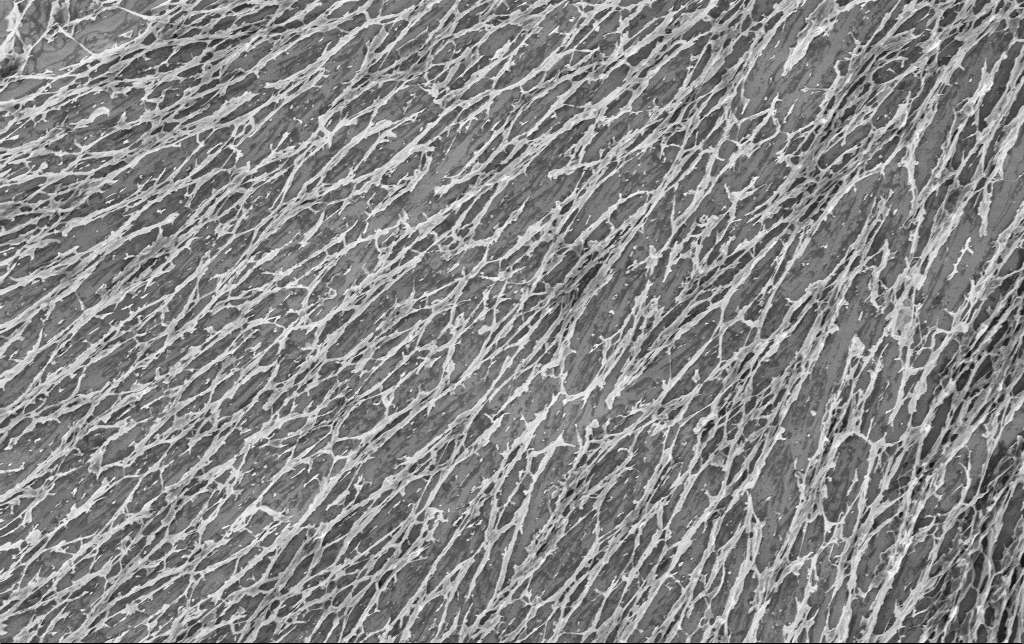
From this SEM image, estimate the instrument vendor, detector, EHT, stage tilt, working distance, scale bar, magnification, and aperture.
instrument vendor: Zeiss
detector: InLens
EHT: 3 kV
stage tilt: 0°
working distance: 3.4 mm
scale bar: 10000 nm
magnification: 4.85 K X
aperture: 30 µm